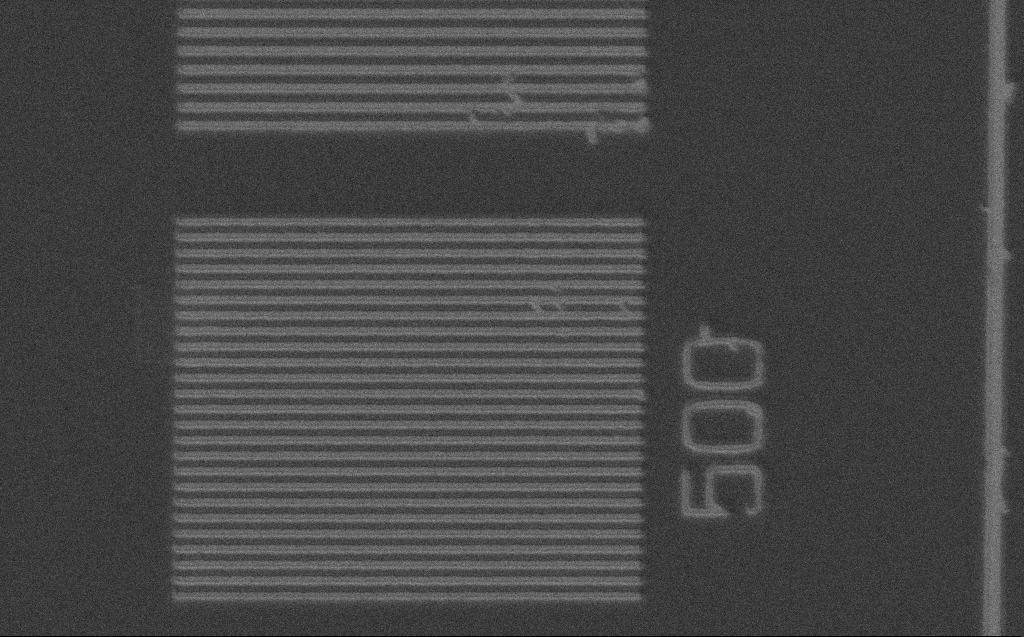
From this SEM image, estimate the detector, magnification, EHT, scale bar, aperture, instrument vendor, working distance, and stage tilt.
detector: SE2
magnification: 5.81 K X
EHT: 3 kV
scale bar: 10000 nm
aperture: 30 µm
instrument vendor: Zeiss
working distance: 4 mm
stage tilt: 0°